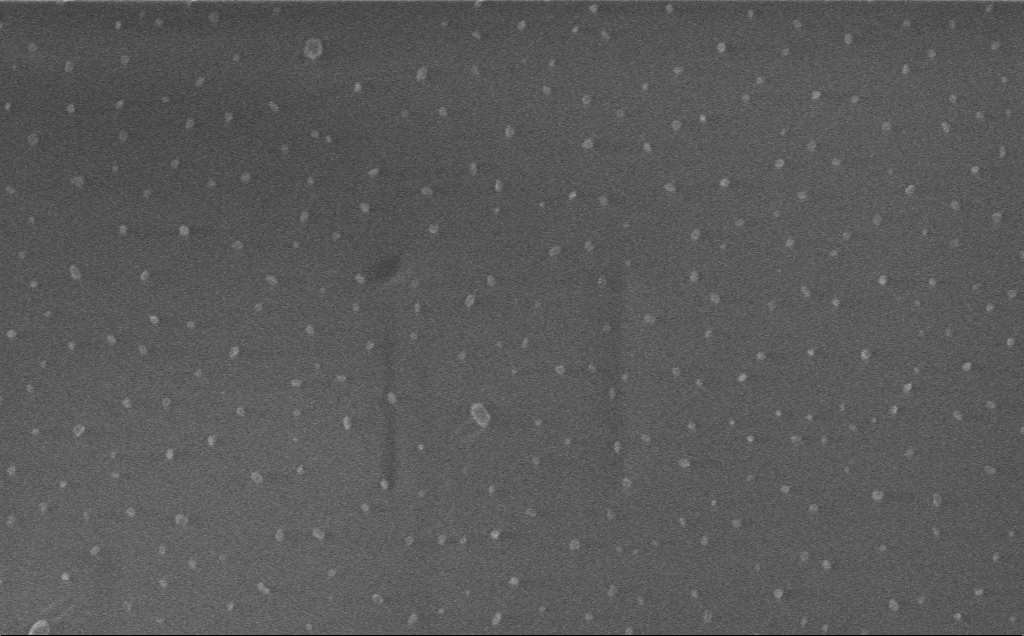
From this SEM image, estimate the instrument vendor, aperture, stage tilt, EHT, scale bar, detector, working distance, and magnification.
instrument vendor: Zeiss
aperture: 30 µm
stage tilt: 0°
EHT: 1 kV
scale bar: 1000 nm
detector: InLens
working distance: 3 mm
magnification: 45.29 K X